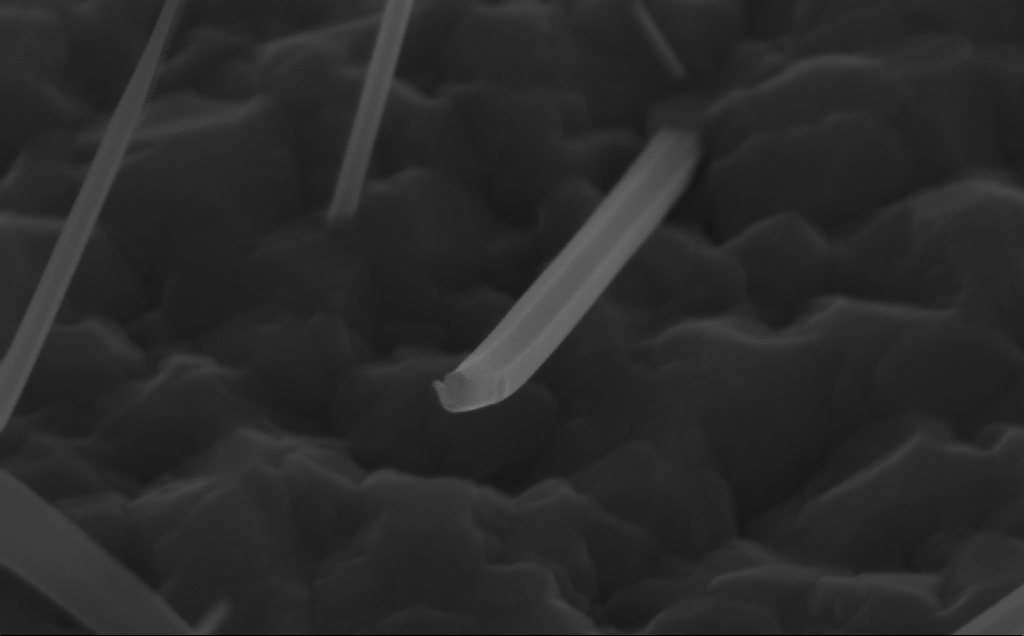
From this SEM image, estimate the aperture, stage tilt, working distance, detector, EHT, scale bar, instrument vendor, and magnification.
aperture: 30 µm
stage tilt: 45°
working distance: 5 mm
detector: InLens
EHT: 10 kV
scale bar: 200 nm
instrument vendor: Zeiss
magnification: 185.71 K X